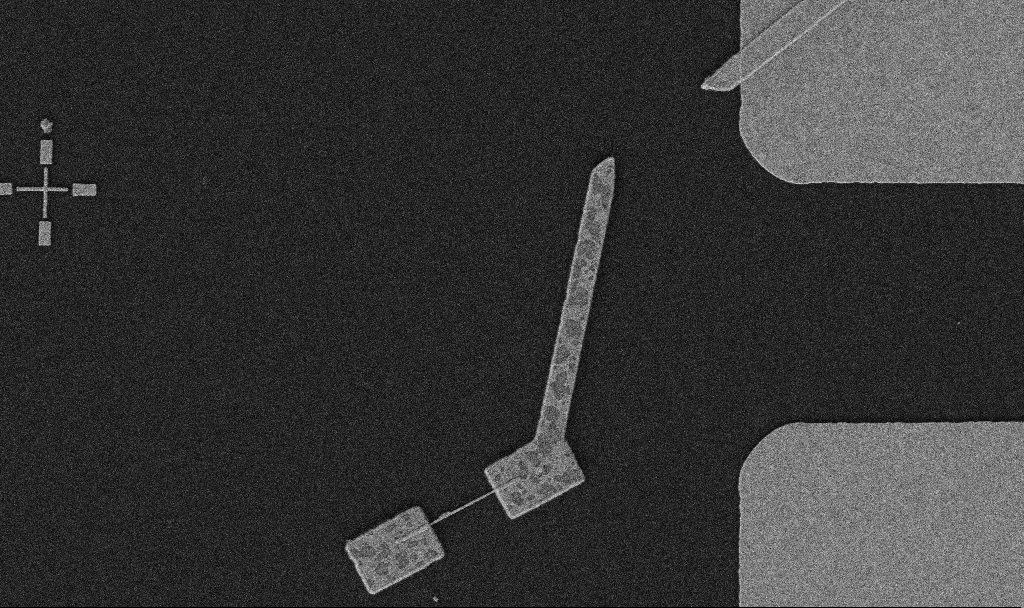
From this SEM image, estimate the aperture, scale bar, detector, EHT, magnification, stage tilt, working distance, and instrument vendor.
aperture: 30 µm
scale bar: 2000 nm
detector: SE2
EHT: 5 kV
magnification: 10 K X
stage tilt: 0°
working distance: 10.7 mm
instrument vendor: Zeiss